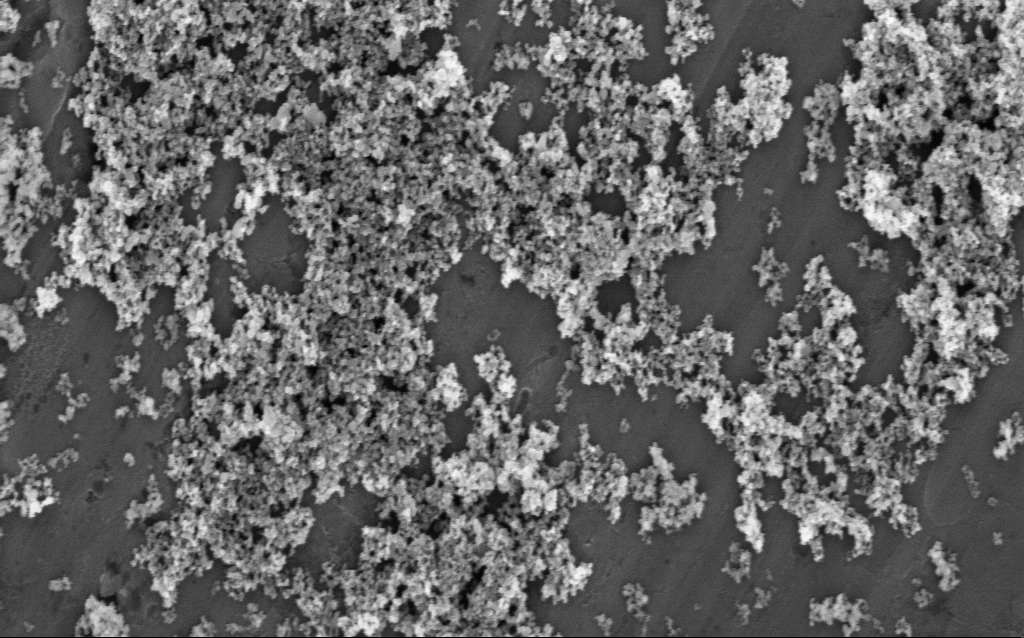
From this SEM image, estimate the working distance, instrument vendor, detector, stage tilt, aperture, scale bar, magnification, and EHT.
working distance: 9.6 mm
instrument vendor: Zeiss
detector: InLens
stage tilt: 0°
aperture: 30 µm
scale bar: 1000 nm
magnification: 46.99 K X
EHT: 5 kV